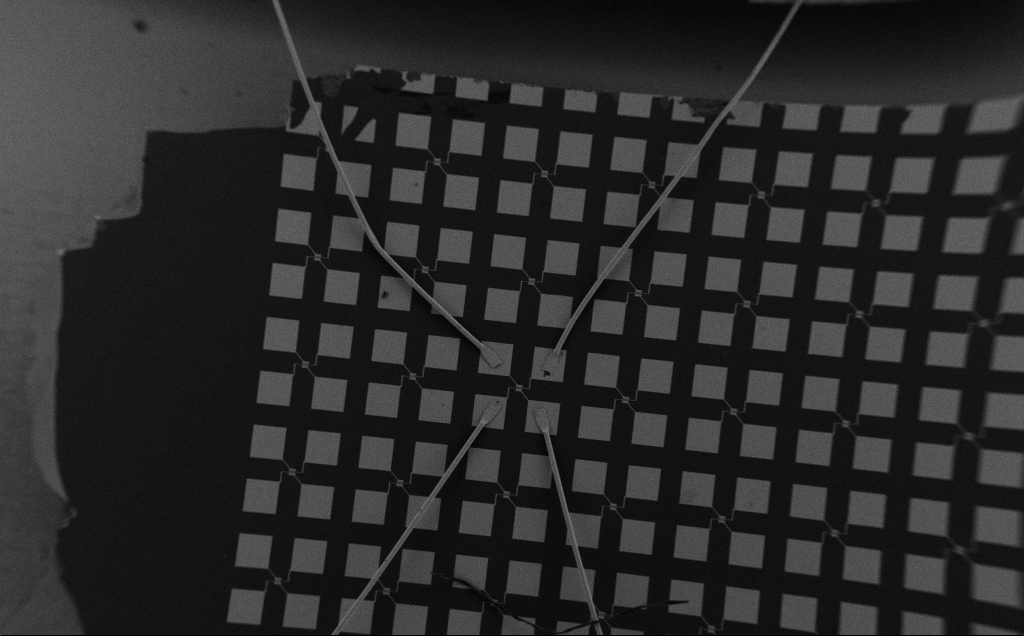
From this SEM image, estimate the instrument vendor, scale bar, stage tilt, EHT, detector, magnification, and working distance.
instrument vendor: Zeiss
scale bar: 200000 nm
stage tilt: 0°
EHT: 5 kV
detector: SE2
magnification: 0.083 K X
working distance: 5 mm